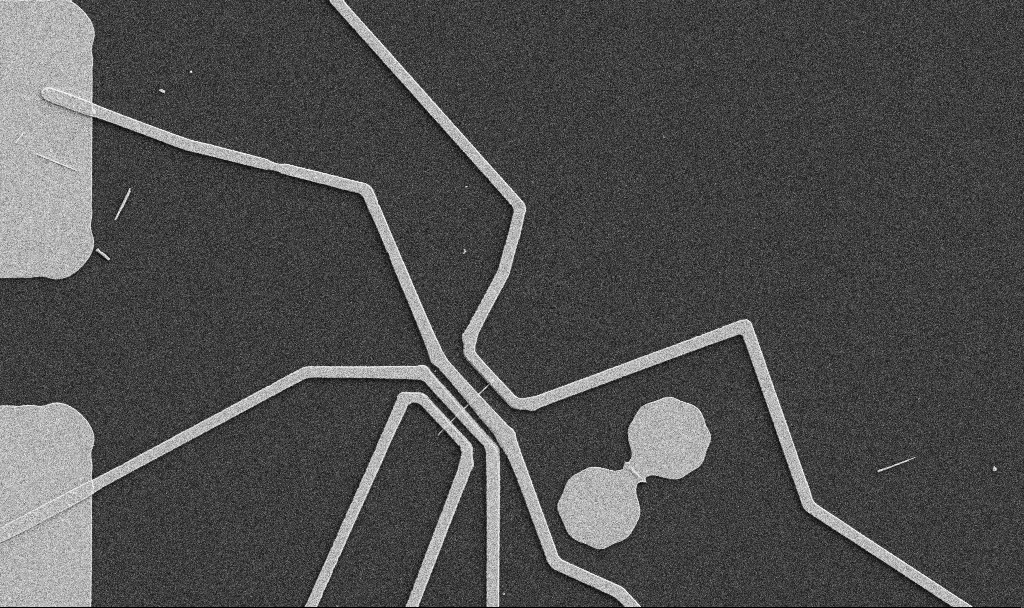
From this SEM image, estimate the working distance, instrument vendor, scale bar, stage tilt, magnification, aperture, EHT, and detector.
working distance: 10.7 mm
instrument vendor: Zeiss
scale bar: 10000 nm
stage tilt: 0°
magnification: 5 K X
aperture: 30 µm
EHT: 5 kV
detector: SE2